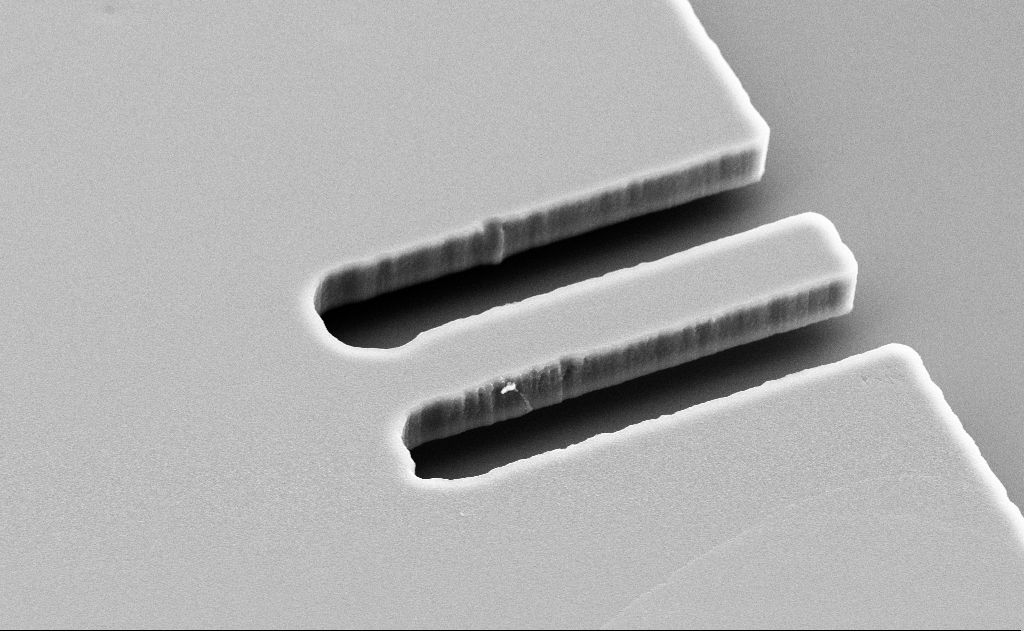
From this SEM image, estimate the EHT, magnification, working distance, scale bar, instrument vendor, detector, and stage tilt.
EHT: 10 kV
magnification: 7.64 K X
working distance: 11 mm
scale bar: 2000 nm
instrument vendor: Zeiss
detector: SE2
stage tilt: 46°